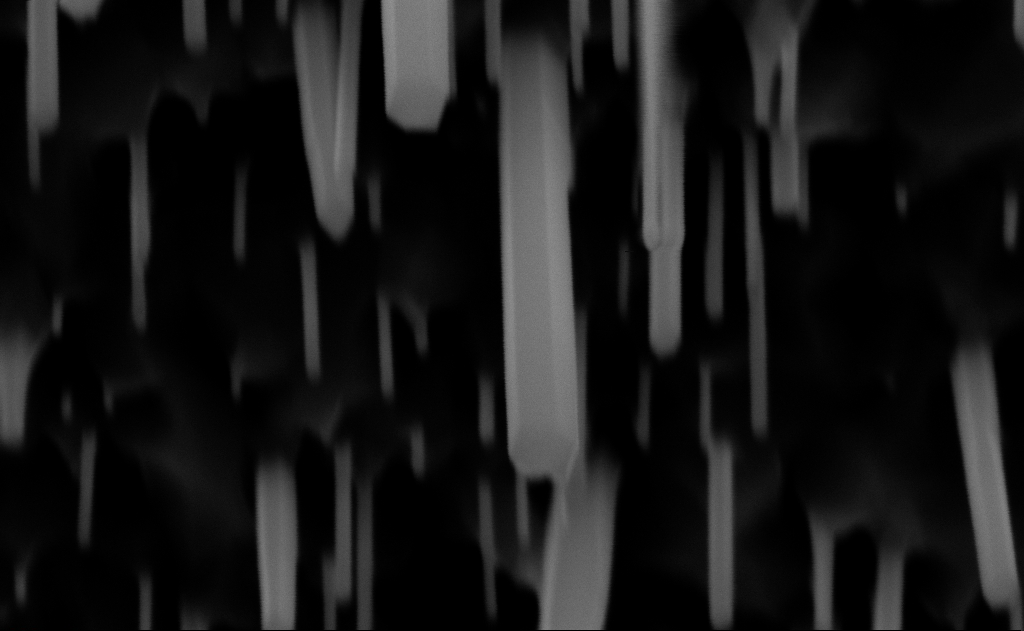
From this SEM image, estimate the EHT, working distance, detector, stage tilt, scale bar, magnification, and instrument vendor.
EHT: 10 kV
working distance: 9 mm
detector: InLens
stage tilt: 0°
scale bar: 200 nm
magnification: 150 K X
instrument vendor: Zeiss